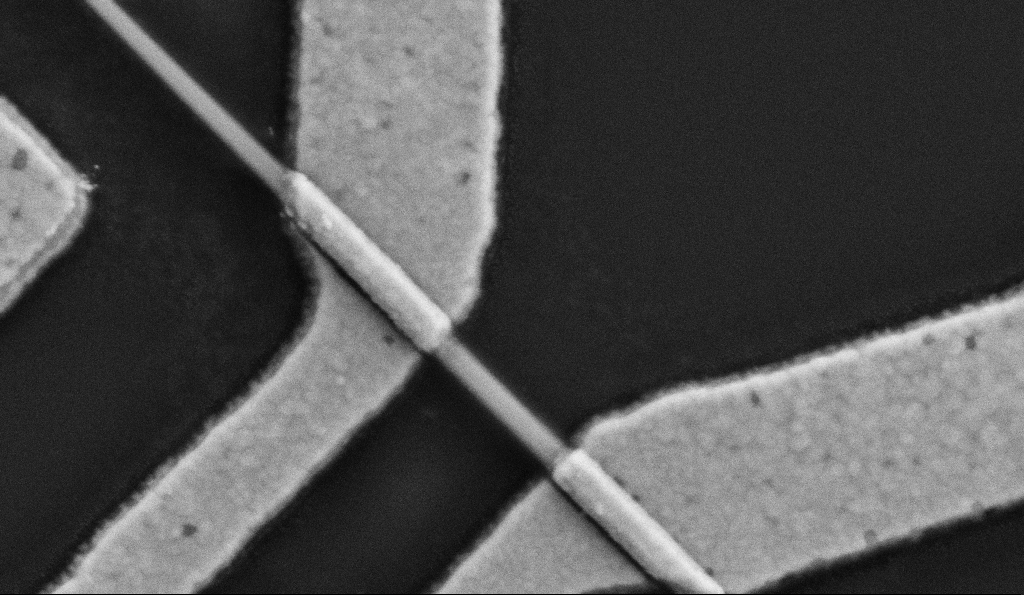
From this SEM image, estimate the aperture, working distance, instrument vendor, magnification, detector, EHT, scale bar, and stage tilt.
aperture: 30 µm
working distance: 9.6 mm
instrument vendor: Zeiss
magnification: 100 K X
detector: SE2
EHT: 5 kV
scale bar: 200 nm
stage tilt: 0°